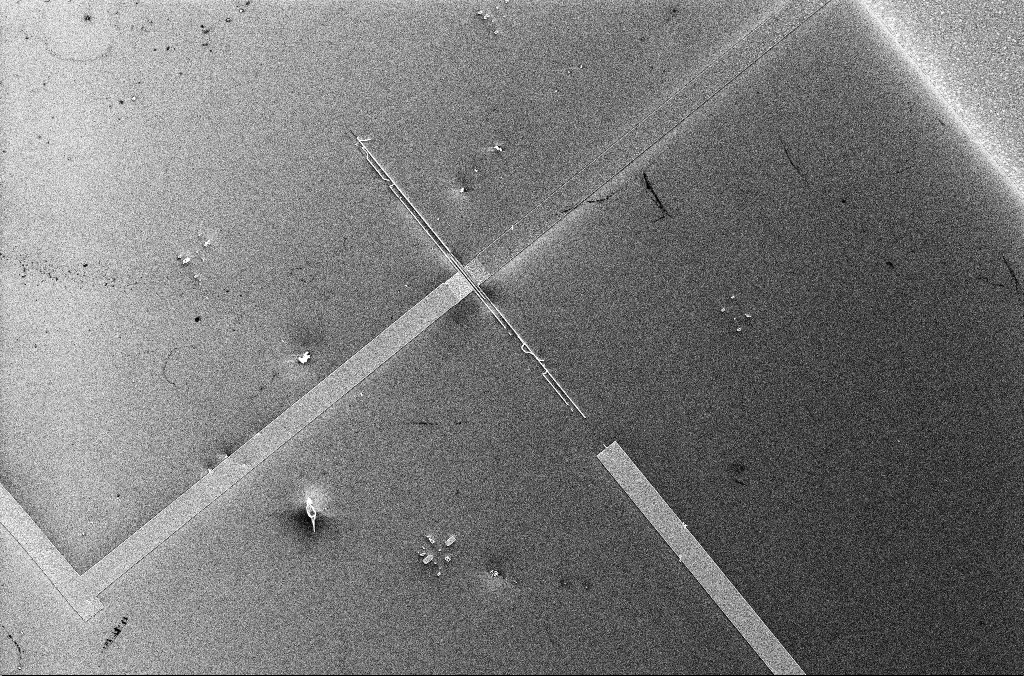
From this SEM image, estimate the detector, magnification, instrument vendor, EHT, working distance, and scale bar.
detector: InLens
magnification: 1.79 K X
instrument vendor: Zeiss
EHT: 5 kV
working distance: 3.3 mm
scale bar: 20000 nm